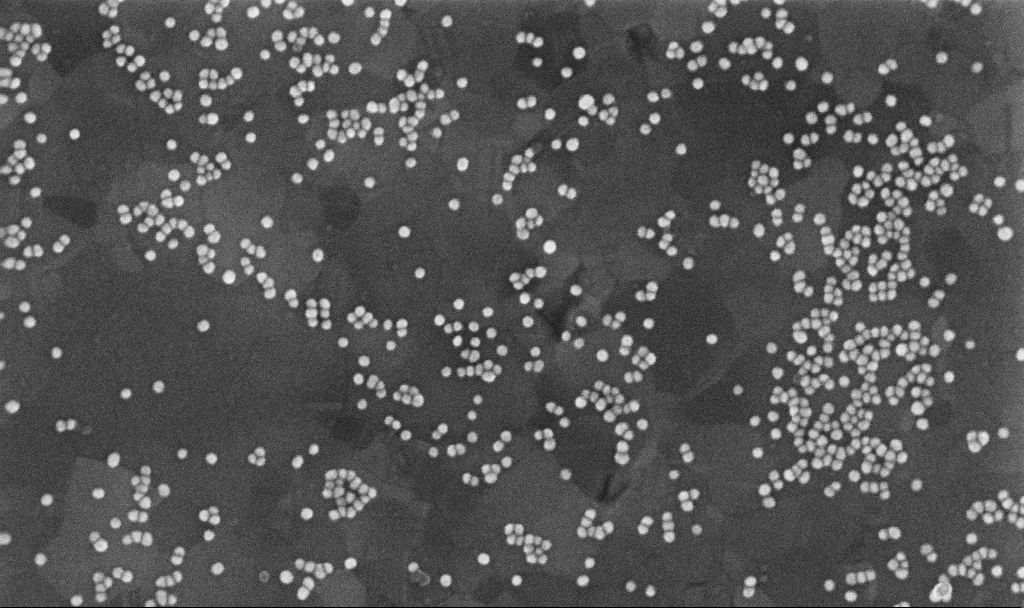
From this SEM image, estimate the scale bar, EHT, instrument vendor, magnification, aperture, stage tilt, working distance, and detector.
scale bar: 200 nm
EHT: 10 kV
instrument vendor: Zeiss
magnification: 200 K X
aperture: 30 µm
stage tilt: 0°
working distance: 3.7 mm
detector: InLens